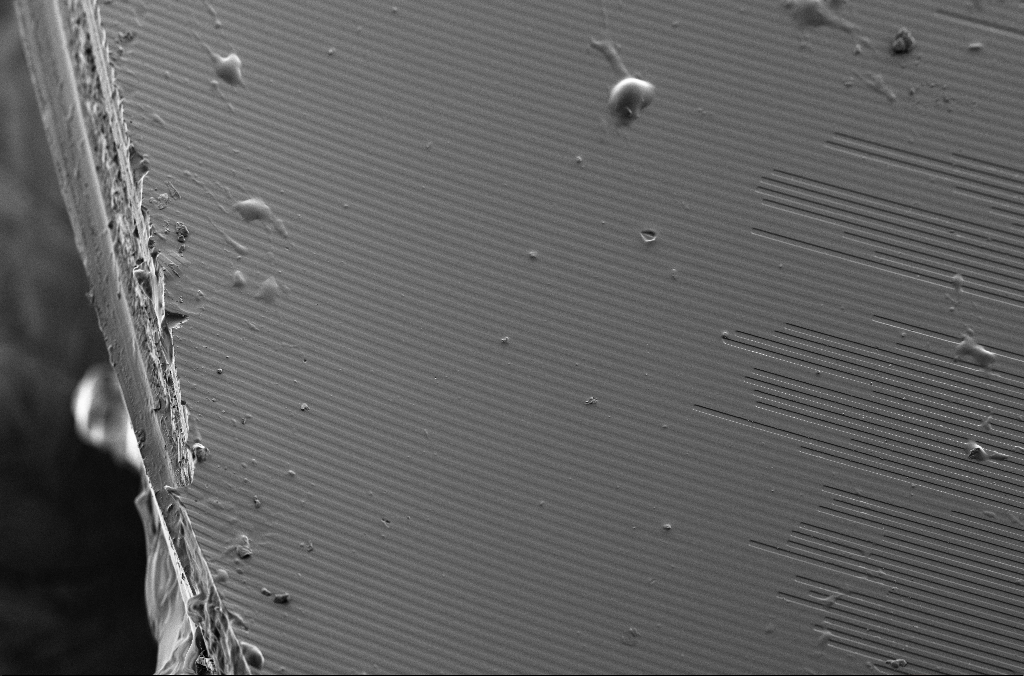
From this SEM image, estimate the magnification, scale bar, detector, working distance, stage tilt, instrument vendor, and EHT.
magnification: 0.2 K X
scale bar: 100000 nm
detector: SE2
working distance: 7.2 mm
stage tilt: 40°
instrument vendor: Zeiss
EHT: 5 kV